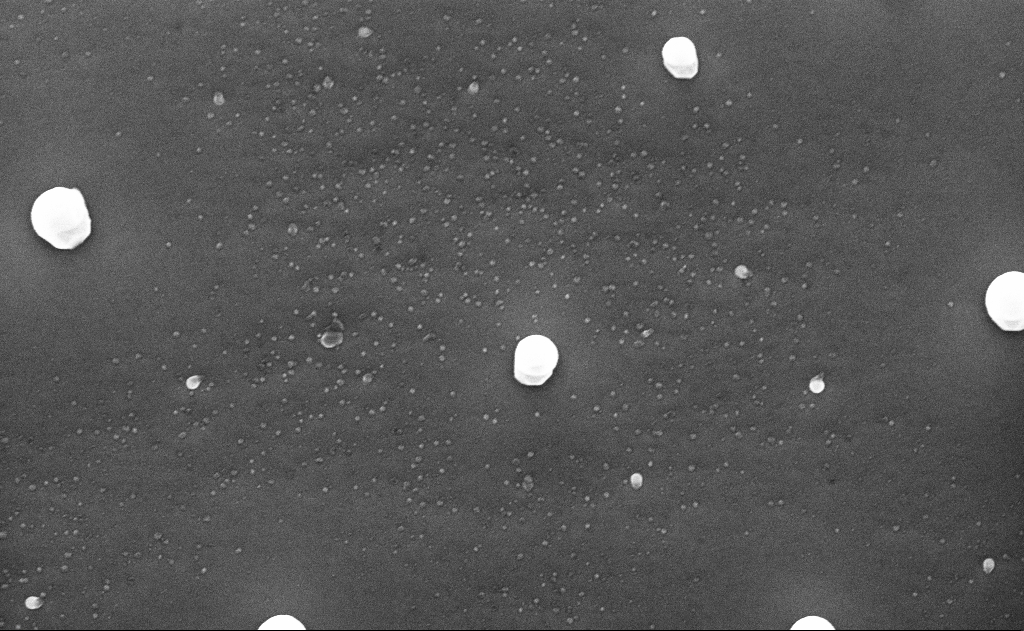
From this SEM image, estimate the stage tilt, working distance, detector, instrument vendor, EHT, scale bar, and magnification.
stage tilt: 0°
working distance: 10 mm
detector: InLens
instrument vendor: Zeiss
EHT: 10 kV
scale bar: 1000 nm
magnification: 50 K X